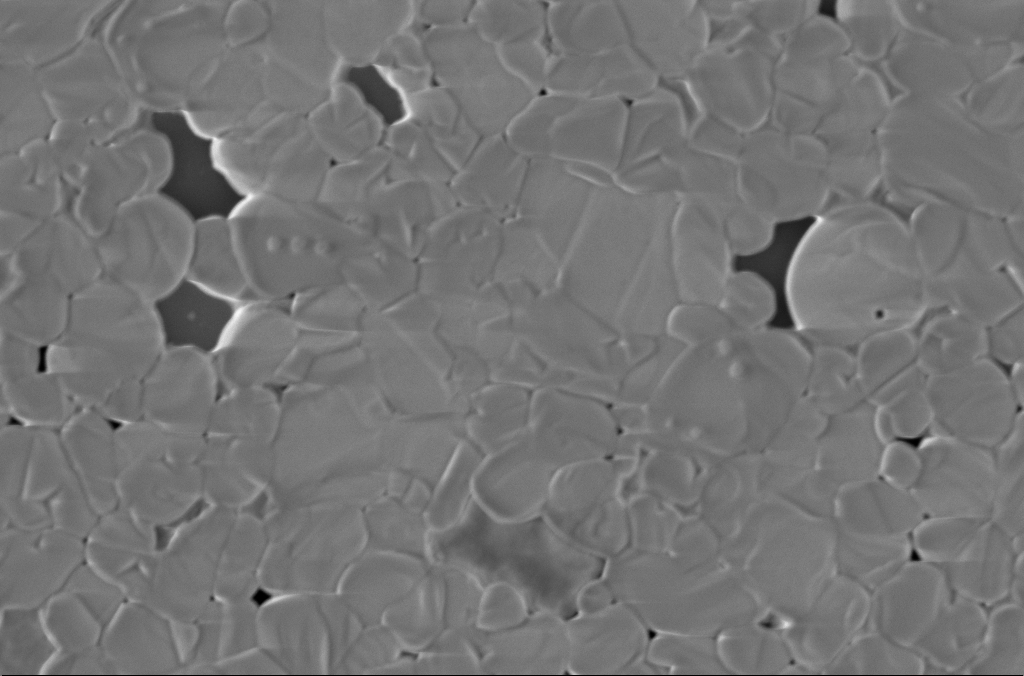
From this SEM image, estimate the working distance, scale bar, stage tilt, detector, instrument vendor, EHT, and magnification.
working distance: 3 mm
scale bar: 1000 nm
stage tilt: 0°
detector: InLens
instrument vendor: Zeiss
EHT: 2 kV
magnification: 50 K X